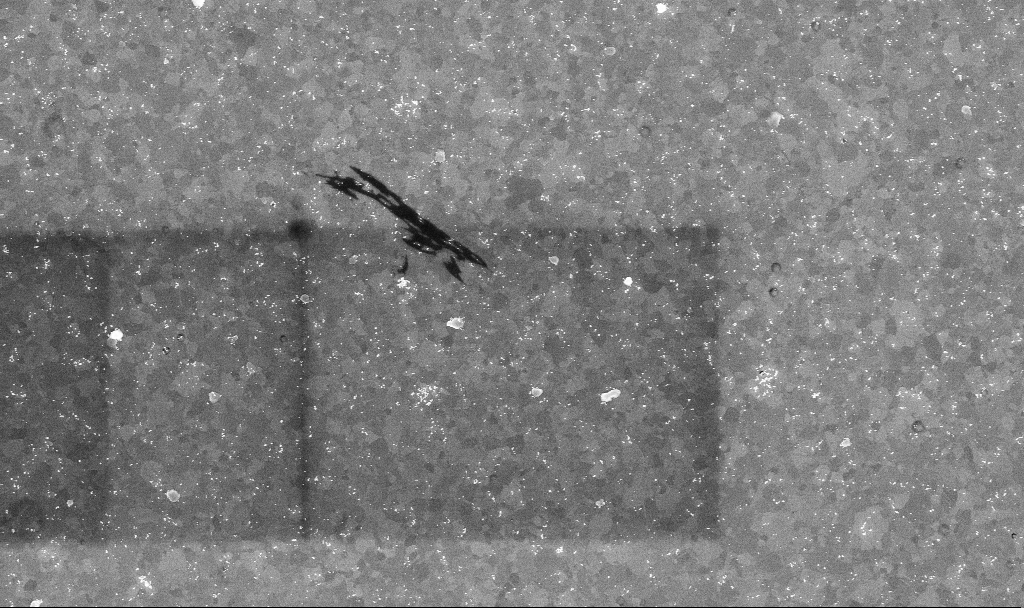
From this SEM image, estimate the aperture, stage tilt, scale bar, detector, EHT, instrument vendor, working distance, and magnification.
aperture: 30 µm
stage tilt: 0°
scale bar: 1000 nm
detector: InLens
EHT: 10 kV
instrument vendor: Zeiss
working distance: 3.4 mm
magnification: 20.42 K X